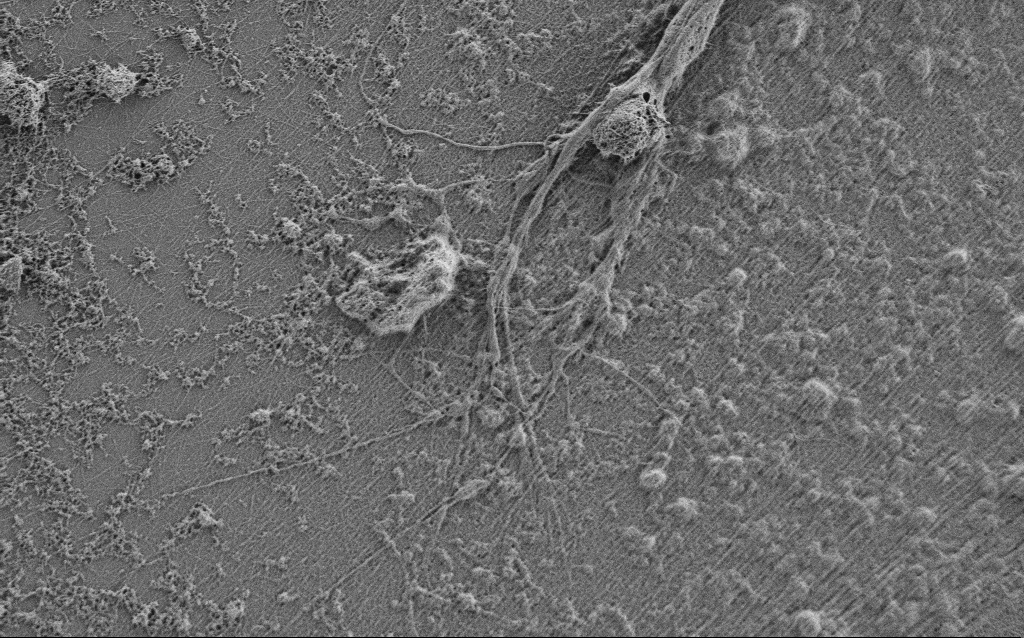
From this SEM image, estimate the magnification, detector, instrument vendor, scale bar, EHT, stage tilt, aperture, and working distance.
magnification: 7.5 K X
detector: SE2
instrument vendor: Zeiss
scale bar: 2000 nm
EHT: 0.9 kV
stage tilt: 0°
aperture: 30 µm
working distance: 4 mm